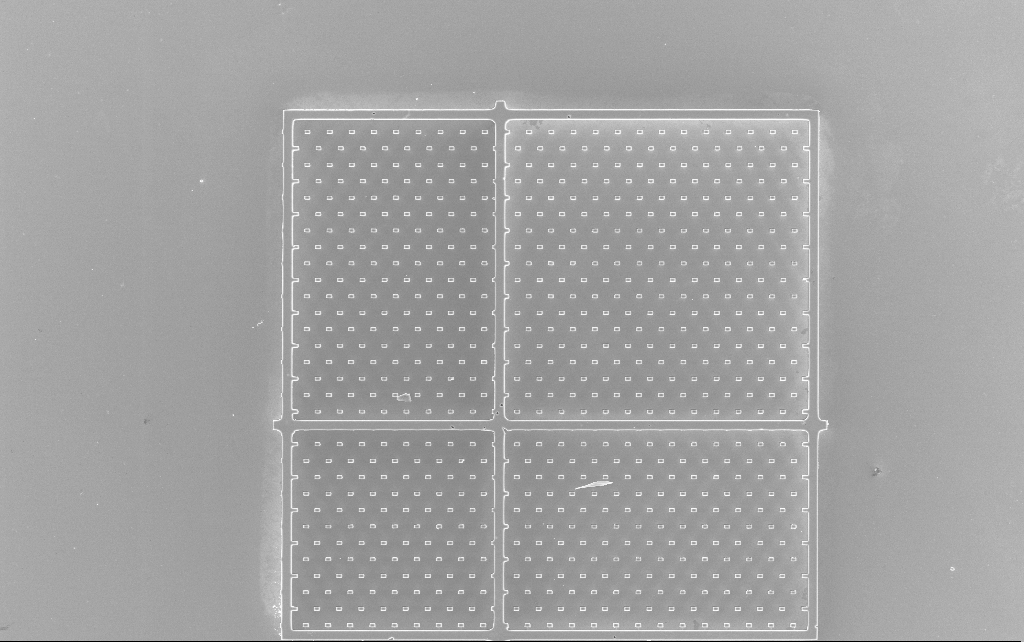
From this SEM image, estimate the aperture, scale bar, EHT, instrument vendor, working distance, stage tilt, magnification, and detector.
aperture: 30 µm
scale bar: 100000 nm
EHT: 10 kV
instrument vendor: Zeiss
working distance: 2.5 mm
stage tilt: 0°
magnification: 0.676 K X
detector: InLens